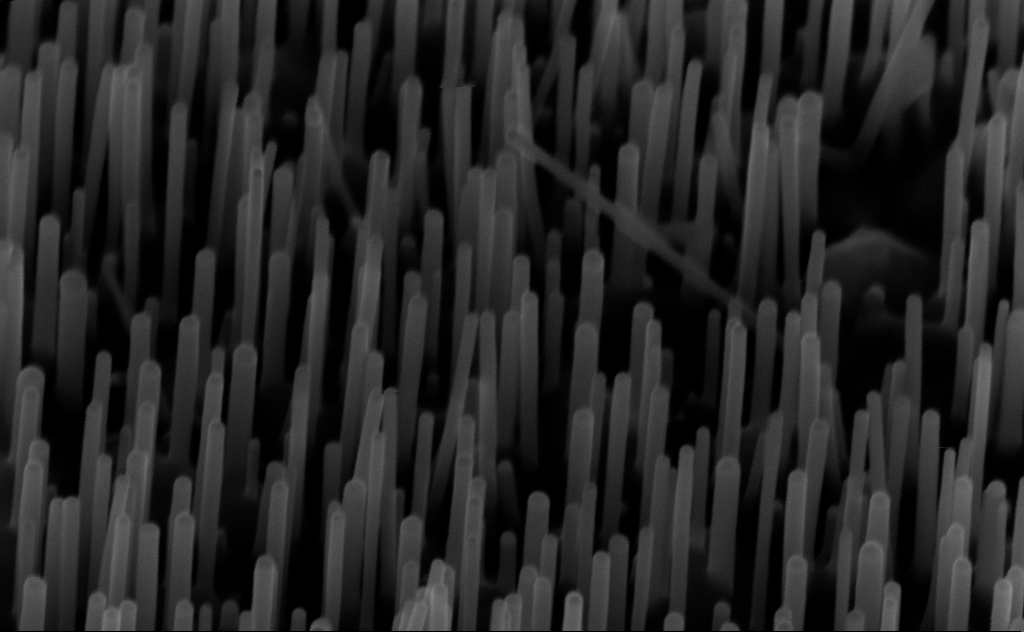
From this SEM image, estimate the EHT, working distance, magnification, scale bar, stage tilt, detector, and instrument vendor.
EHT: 10 kV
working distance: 6 mm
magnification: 150 K X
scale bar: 200 nm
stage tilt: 45°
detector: InLens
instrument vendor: Zeiss